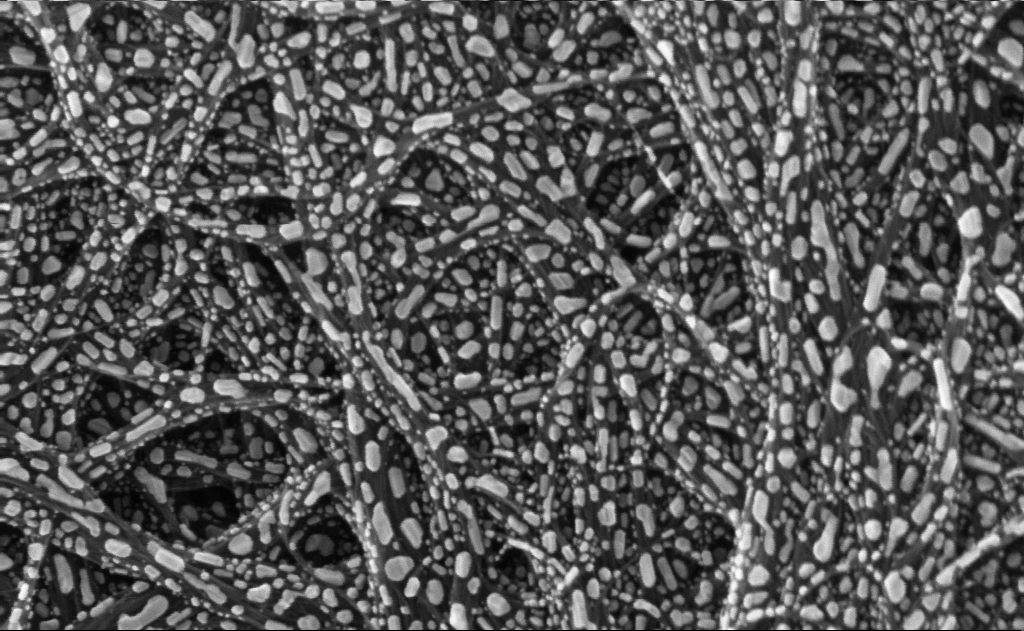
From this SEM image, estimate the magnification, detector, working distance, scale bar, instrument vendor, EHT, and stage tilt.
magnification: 269.48 K X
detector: InLens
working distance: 3 mm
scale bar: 200 nm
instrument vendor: Zeiss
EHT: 10 kV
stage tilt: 0°